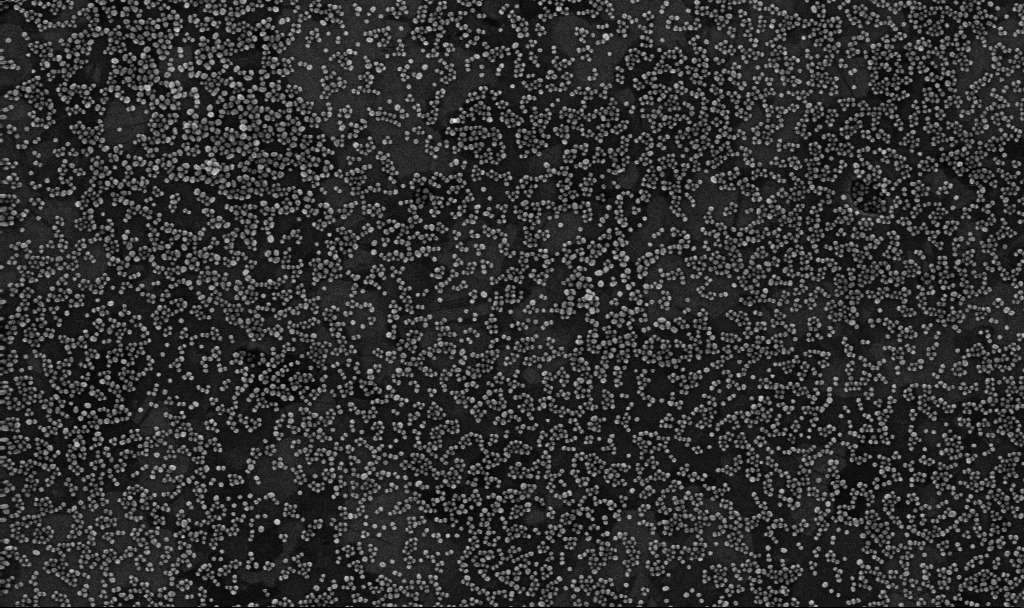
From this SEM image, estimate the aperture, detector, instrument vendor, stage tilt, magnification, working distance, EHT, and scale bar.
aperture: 30 µm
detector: InLens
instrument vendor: Zeiss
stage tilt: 0°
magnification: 100 K X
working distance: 3.3 mm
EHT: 10 kV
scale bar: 200 nm